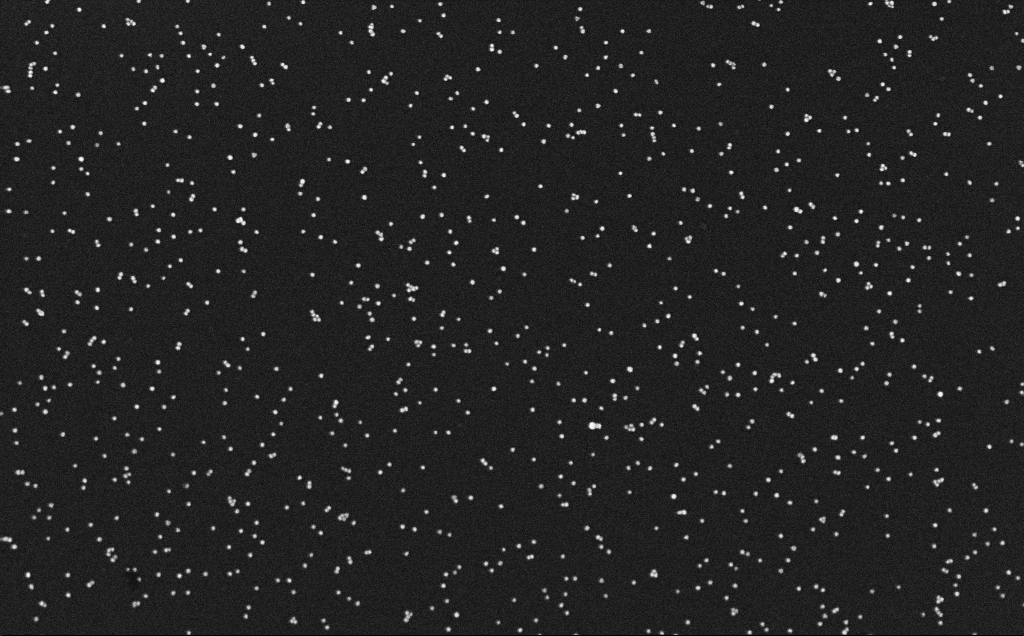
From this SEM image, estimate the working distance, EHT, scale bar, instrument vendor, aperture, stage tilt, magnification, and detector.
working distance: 3.1 mm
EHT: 10 kV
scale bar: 200 nm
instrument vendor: Zeiss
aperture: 30 µm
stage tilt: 0°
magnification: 100 K X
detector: InLens